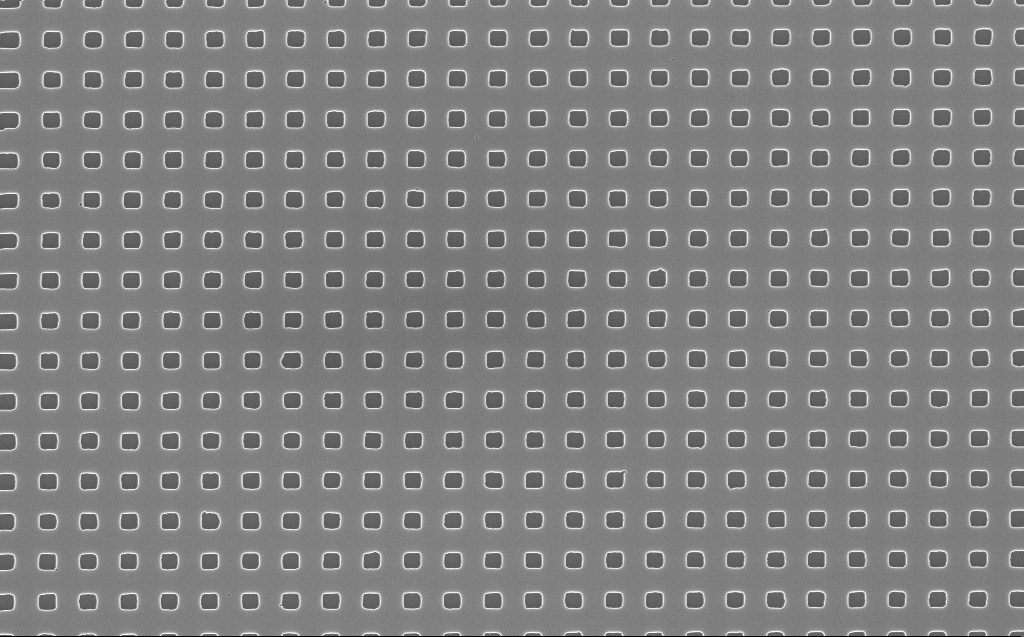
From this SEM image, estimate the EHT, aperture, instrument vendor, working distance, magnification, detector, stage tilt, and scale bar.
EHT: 10 kV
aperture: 30 µm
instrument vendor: Zeiss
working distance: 5 mm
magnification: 30 K X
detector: InLens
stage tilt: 0°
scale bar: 2000 nm